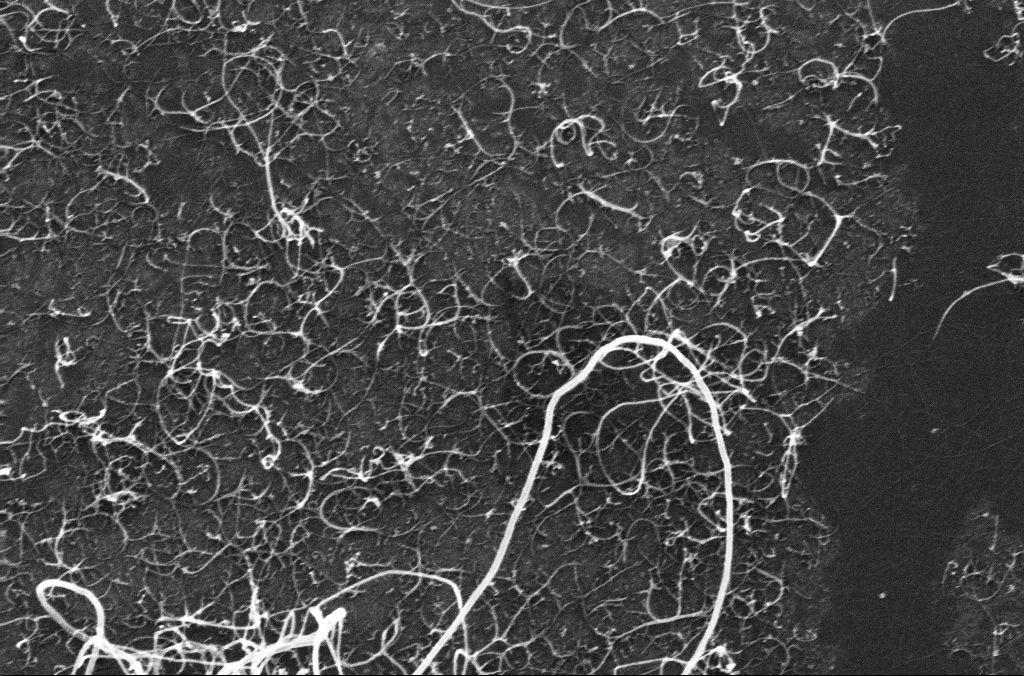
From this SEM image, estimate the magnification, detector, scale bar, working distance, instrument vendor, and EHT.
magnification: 106.01 K X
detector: InLens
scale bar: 200 nm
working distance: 3.2 mm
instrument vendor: Zeiss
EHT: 10 kV